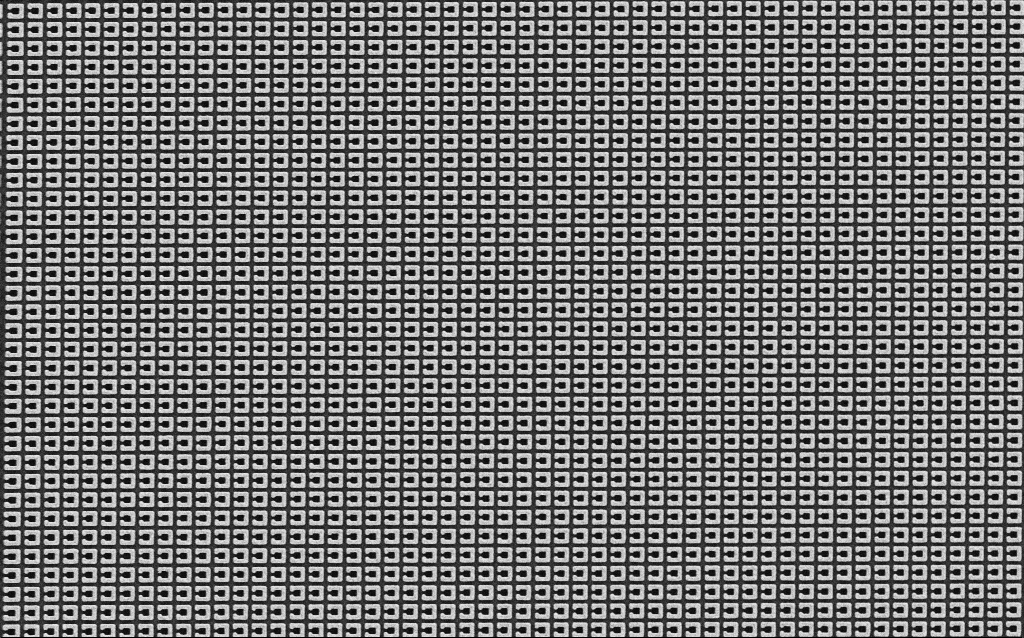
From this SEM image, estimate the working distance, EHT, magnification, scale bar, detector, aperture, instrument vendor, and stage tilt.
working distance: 6 mm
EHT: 5 kV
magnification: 15.1 K X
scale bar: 2000 nm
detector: SE2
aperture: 30 µm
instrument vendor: Zeiss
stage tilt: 0°